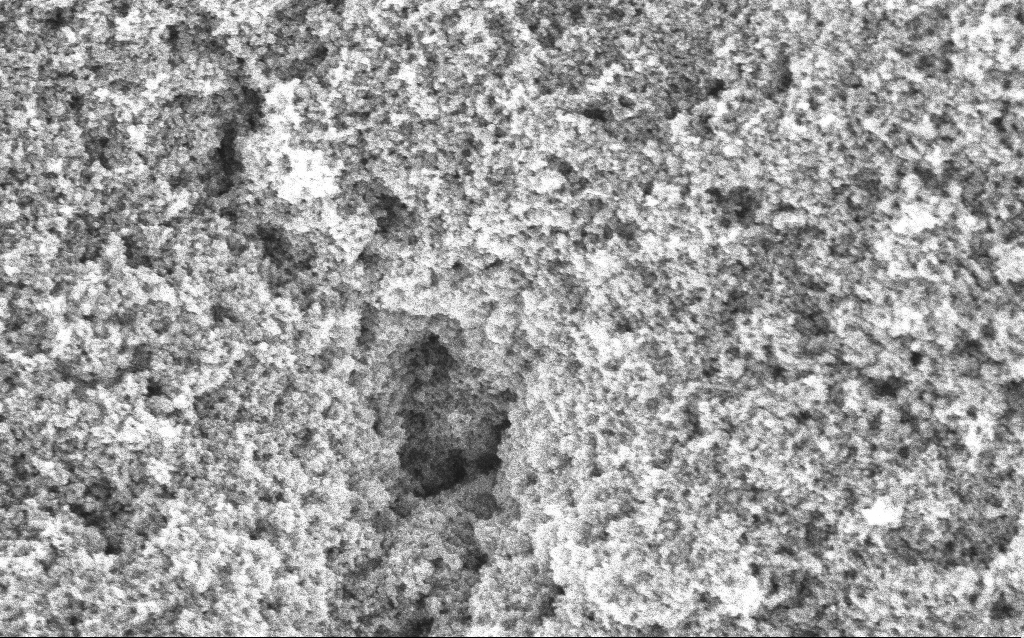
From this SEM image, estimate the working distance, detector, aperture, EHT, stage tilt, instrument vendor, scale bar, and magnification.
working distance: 4.2 mm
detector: InLens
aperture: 30 µm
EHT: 5 kV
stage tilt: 0°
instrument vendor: Zeiss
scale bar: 1000 nm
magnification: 65.04 K X